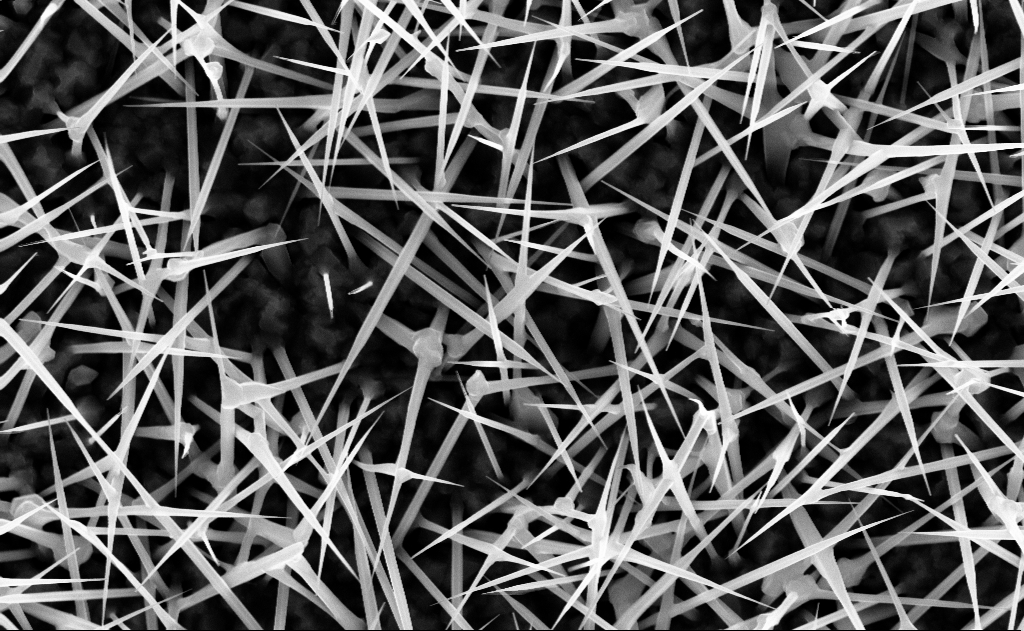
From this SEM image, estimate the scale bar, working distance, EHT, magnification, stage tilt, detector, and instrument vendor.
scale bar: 1000 nm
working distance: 9 mm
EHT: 10 kV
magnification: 40 K X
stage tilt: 0°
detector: InLens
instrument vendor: Zeiss